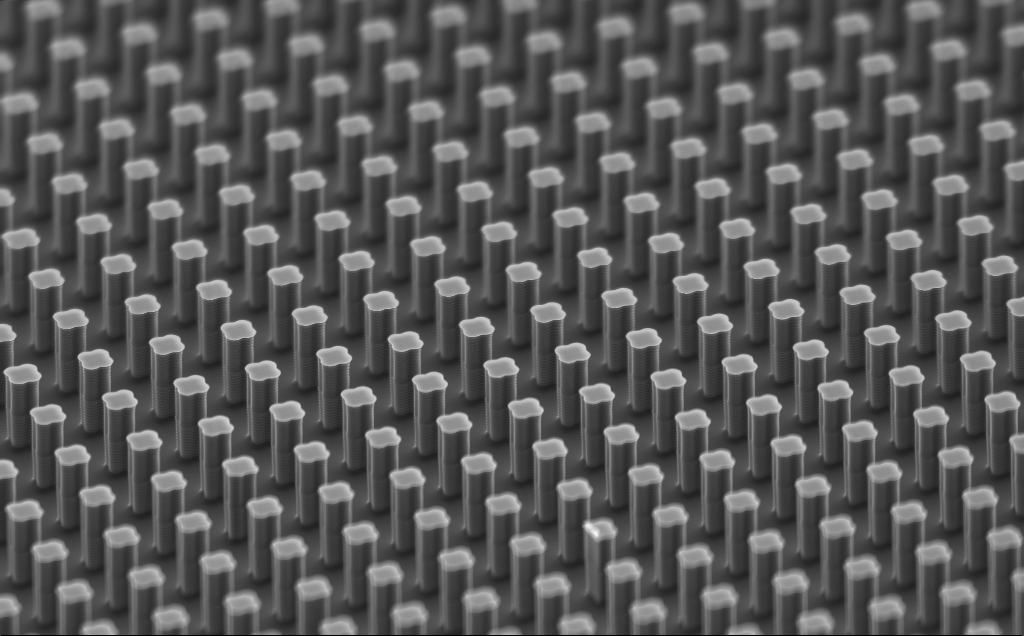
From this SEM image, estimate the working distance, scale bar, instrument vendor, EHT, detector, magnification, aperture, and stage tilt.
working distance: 15 mm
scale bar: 10000 nm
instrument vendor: Zeiss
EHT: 10 kV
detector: SE2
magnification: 2.76 K X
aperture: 120 µm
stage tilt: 59.6°